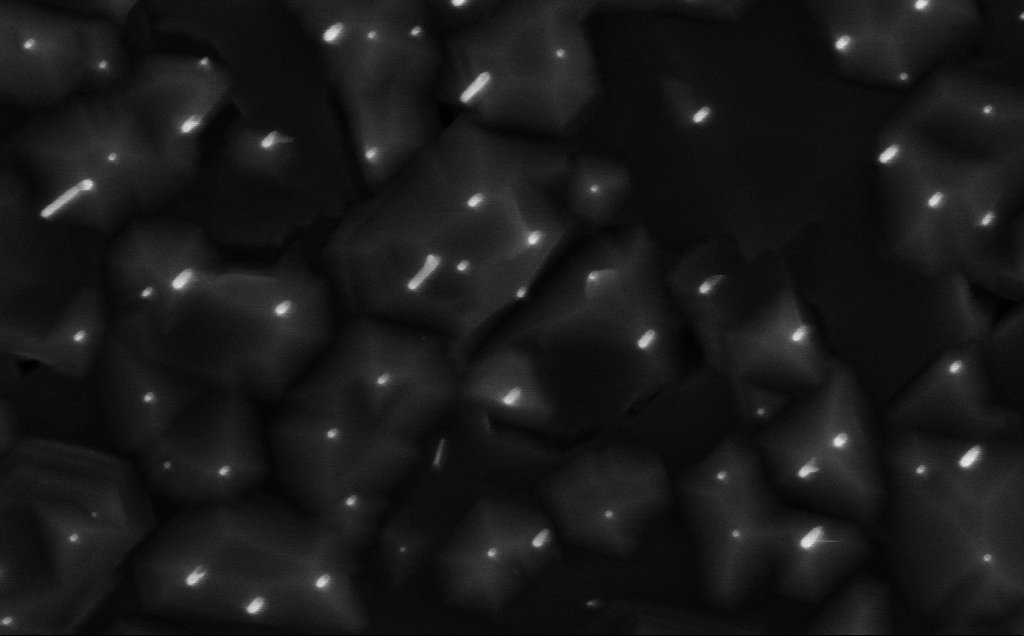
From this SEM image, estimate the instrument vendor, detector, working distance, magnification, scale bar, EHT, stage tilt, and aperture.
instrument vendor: Zeiss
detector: InLens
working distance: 4 mm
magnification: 80 K X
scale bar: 200 nm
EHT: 7 kV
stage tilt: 0°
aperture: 30 µm